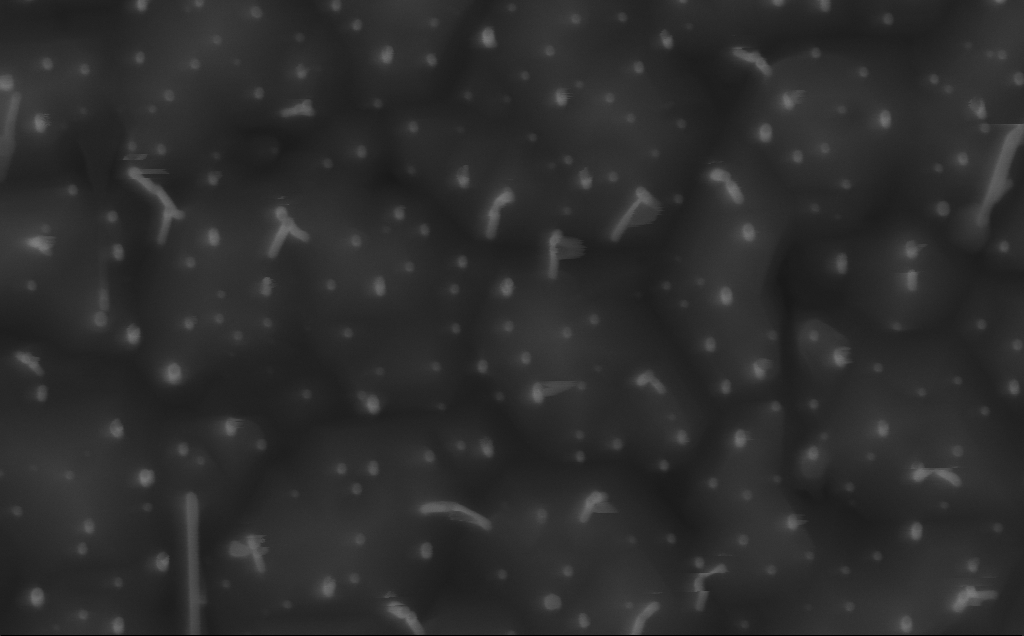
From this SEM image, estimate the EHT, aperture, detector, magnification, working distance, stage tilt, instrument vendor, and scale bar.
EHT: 10 kV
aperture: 30 µm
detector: InLens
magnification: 115.42 K X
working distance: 5 mm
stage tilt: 0°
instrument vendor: Zeiss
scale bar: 200 nm